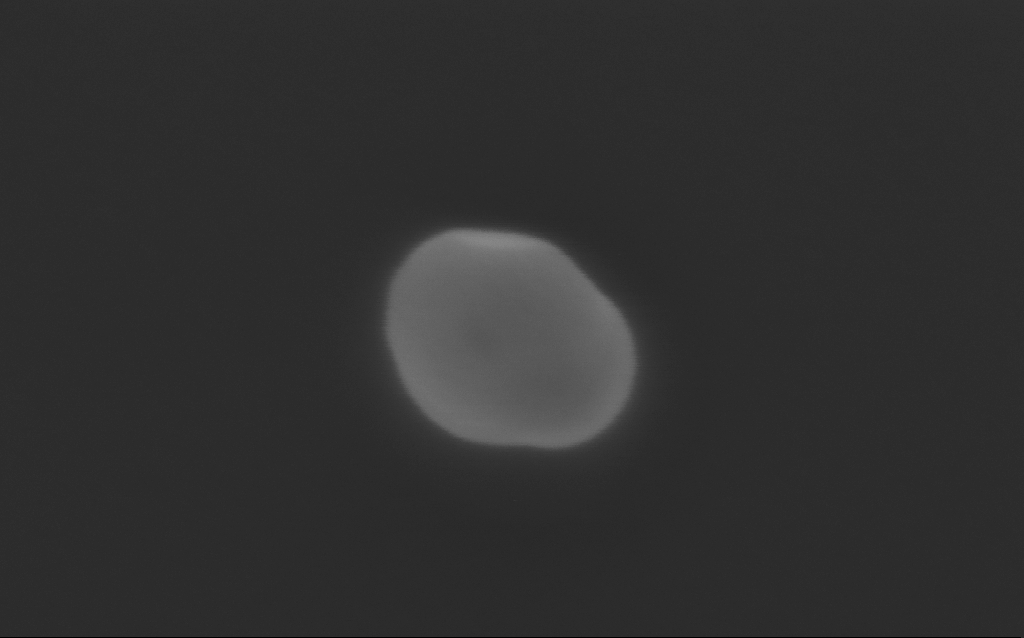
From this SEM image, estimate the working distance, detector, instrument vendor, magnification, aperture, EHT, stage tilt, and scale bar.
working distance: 3 mm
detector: InLens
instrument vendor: Zeiss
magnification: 480.49 K X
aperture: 30 µm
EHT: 3 kV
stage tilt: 0°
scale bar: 100 nm